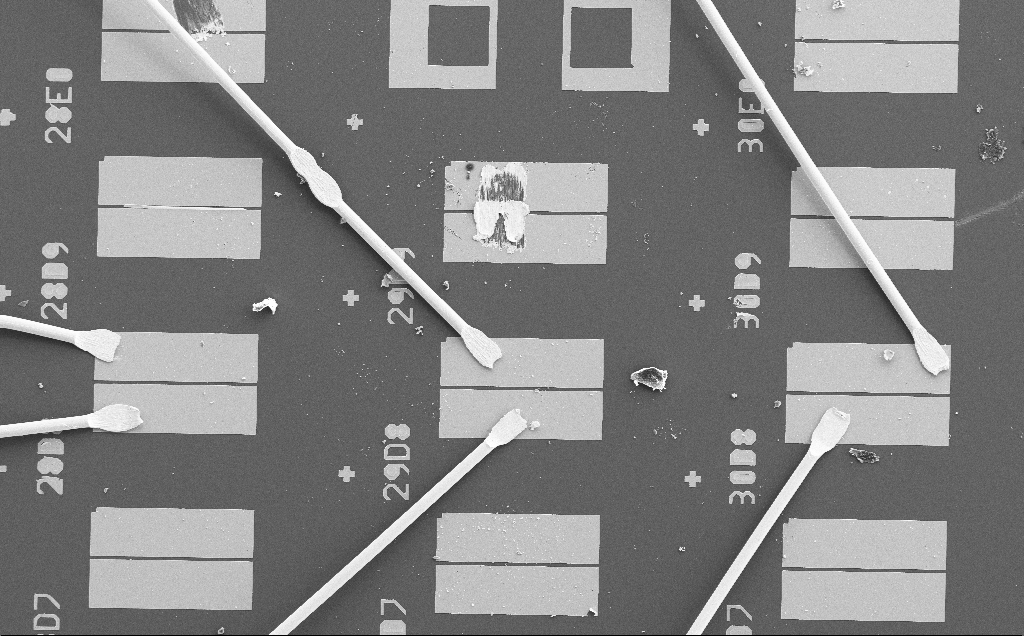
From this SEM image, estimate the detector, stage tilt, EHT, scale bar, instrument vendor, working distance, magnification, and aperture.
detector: SE2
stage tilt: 0°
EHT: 15 kV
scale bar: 200000 nm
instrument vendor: Zeiss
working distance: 10 mm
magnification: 0.186 K X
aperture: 30 µm